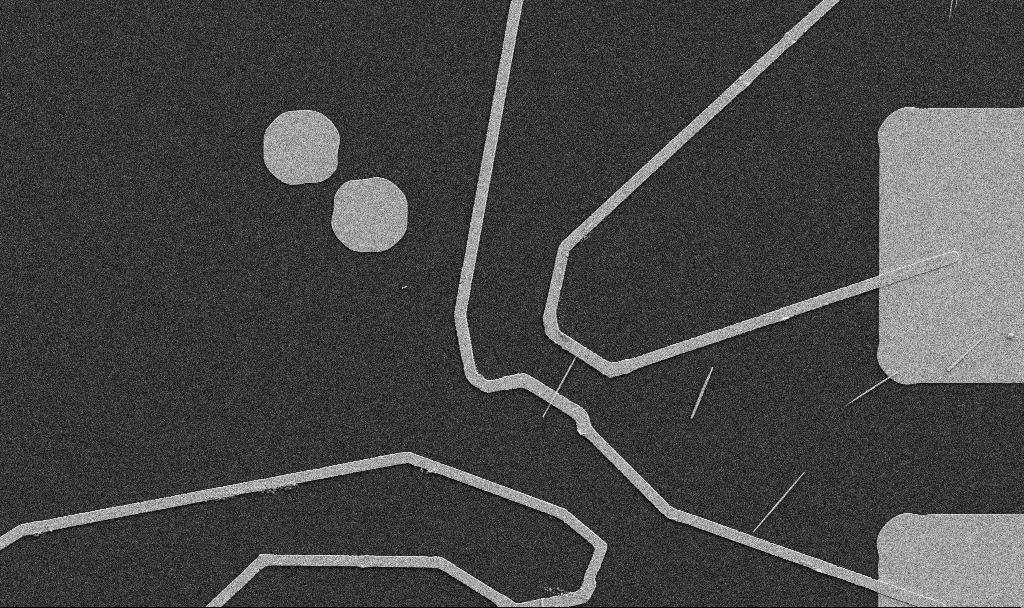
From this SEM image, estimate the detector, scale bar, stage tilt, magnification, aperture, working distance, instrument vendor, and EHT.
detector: SE2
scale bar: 10000 nm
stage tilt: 0°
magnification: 5 K X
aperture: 30 µm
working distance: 10.7 mm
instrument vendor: Zeiss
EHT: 5 kV